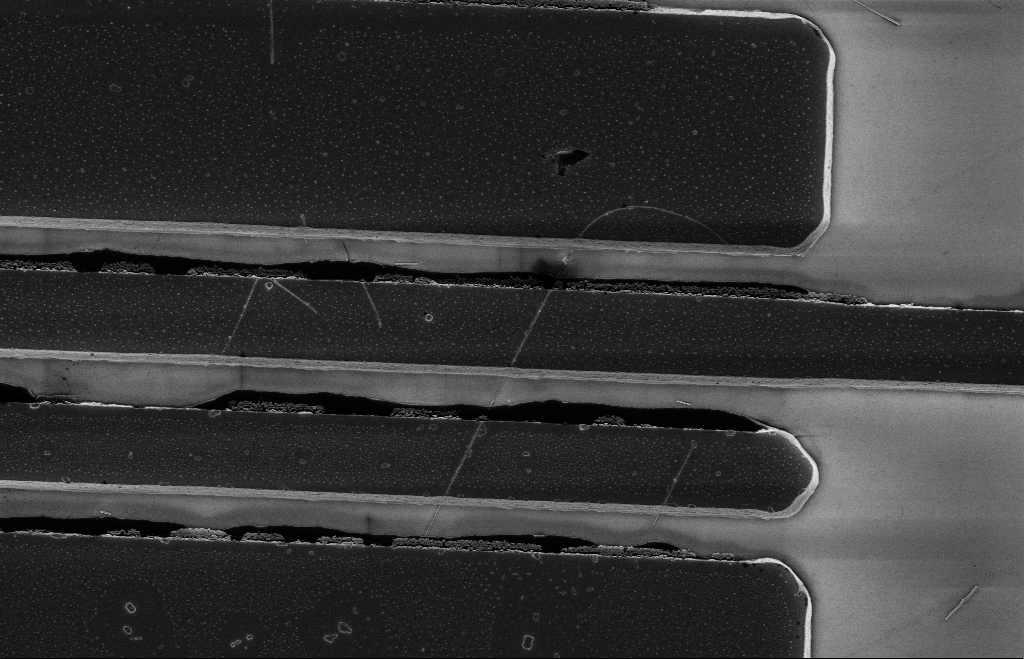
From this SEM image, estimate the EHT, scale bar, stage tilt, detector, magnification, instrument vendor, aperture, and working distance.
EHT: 5 kV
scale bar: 1000 nm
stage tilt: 0°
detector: InLens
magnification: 8.07 K X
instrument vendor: Zeiss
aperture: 20 µm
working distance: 12 mm